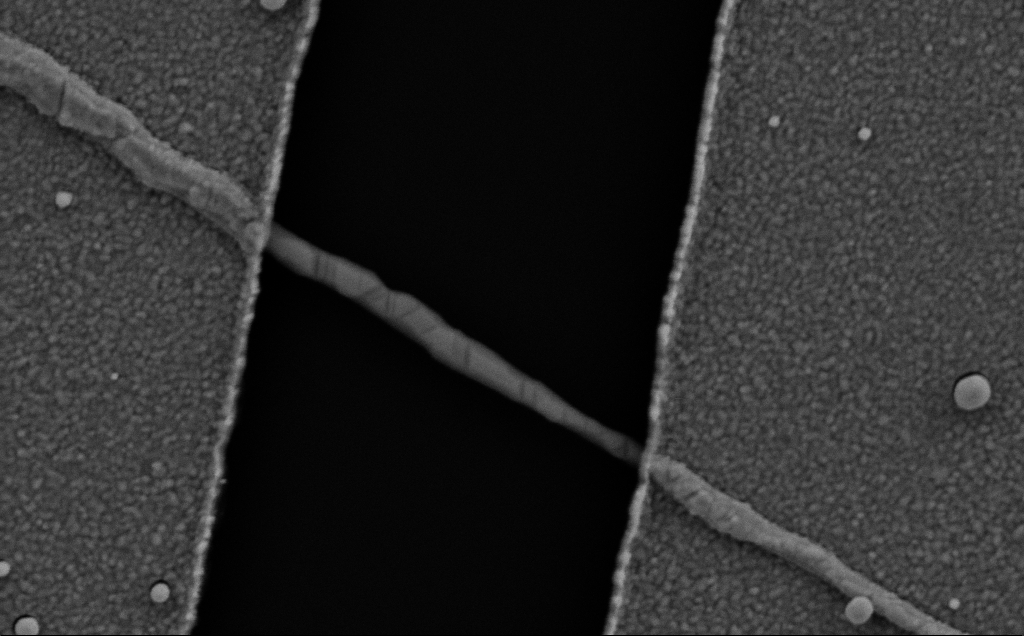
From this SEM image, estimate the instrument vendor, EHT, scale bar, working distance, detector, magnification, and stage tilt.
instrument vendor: Zeiss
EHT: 5 kV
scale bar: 200 nm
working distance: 8 mm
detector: SE2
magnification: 82.35 K X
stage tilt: -0.7°